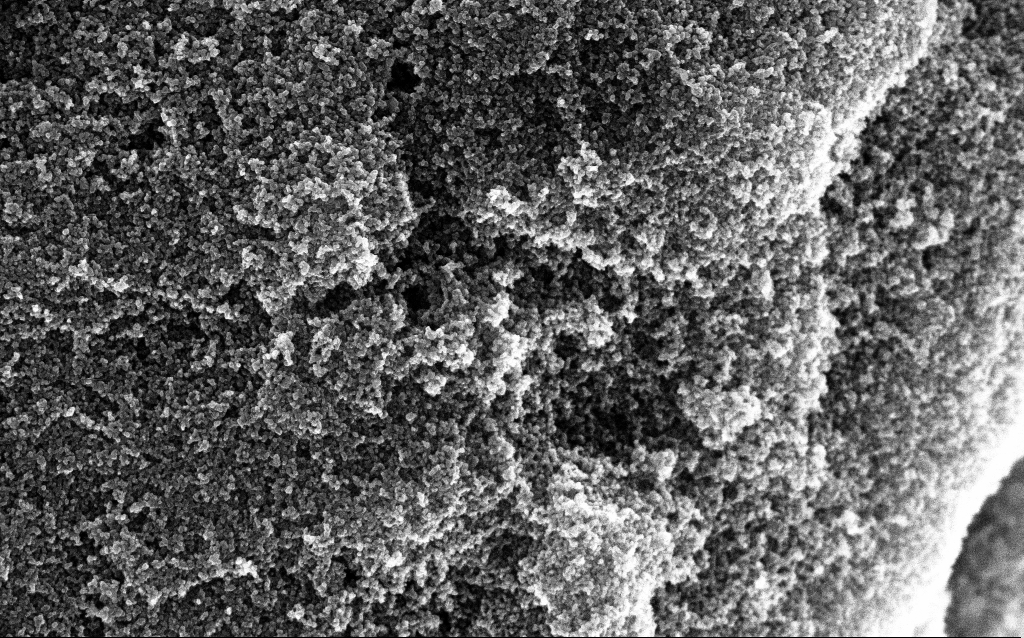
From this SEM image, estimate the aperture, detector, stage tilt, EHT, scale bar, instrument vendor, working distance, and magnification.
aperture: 30 µm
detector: InLens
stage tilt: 0°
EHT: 10 kV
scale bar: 1000 nm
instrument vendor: Zeiss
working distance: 2.8 mm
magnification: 65.04 K X